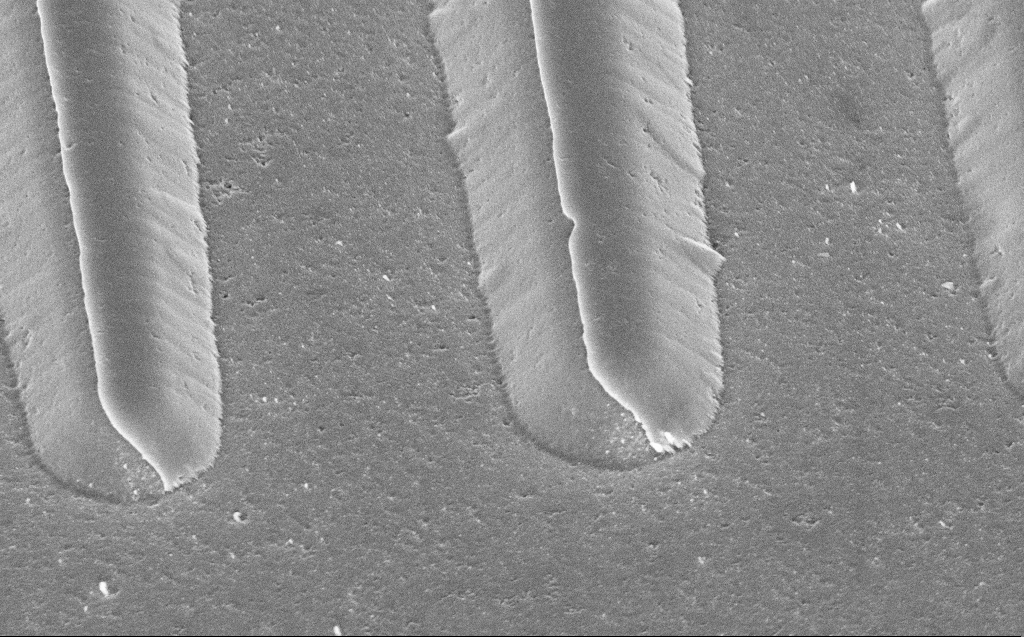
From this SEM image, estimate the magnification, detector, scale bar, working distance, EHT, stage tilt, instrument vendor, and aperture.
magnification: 20.19 K X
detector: InLens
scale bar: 2000 nm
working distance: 11 mm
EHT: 5 kV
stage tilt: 45°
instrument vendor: Zeiss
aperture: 30 µm